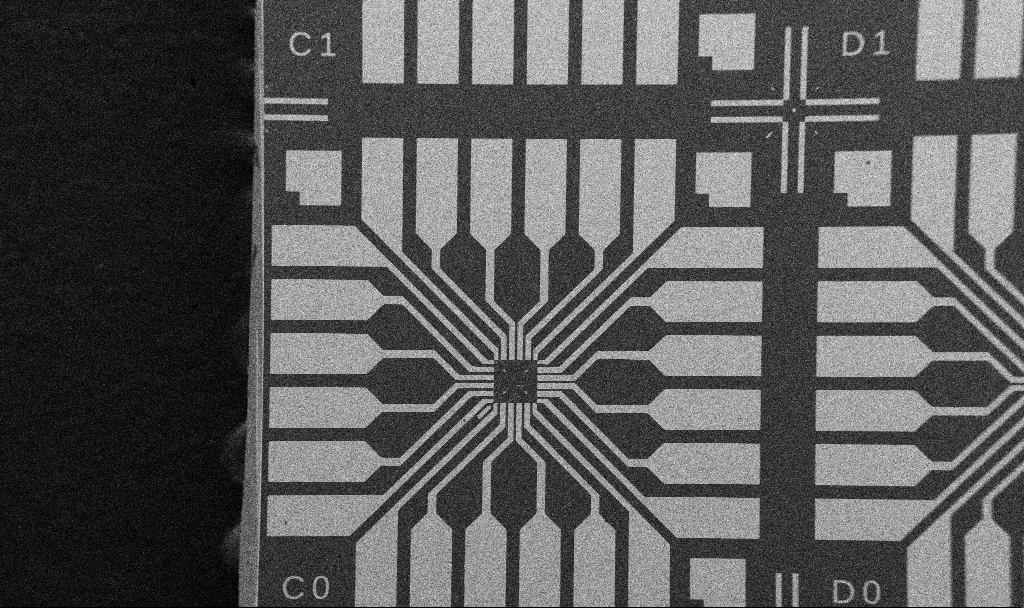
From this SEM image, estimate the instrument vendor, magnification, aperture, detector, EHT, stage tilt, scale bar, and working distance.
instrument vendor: Zeiss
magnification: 0.1 K X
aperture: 30 µm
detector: SE2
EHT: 5 kV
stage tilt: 0°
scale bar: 200000 nm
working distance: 10.7 mm